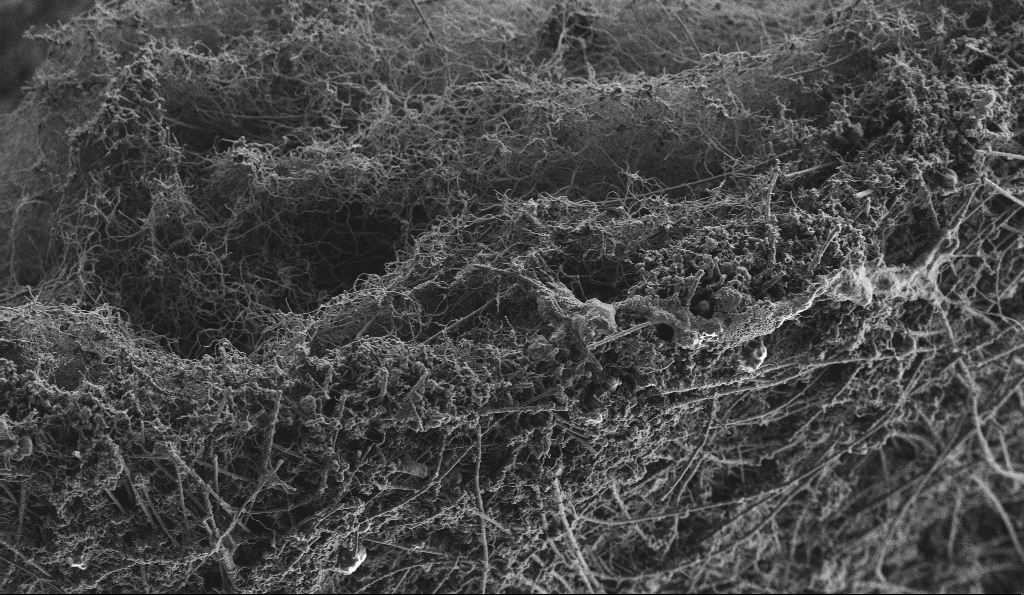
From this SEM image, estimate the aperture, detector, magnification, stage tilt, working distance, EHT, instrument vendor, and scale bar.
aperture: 30 µm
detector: SE2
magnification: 2.5 K X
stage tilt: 0°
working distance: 4.4 mm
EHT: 3 kV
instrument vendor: Zeiss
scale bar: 10000 nm